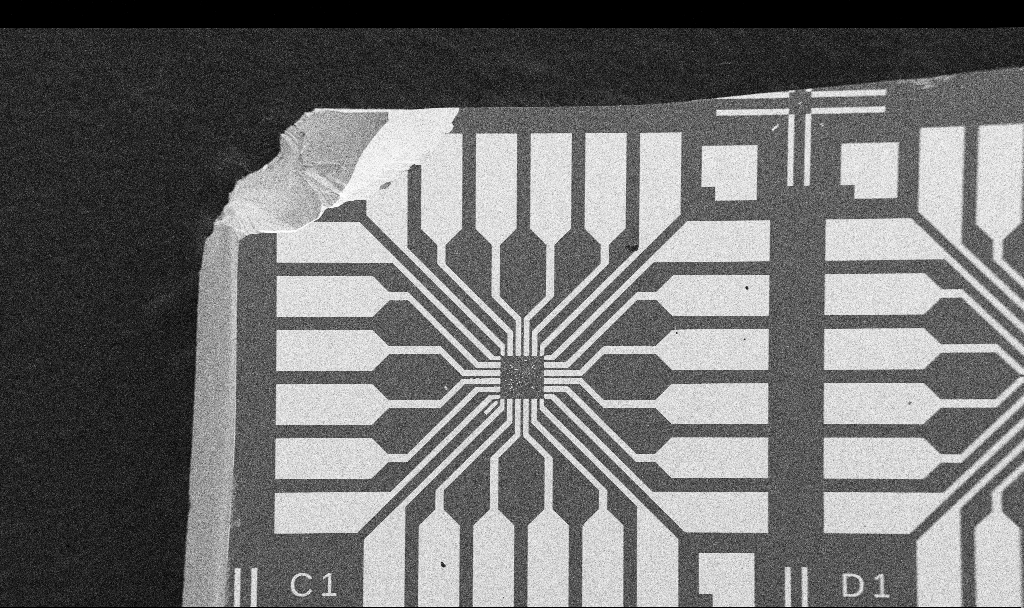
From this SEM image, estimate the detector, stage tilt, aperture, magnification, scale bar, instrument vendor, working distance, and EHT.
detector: SE2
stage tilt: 0°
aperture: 30 µm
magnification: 0.1 K X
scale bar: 200000 nm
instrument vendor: Zeiss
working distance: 10.7 mm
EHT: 5 kV